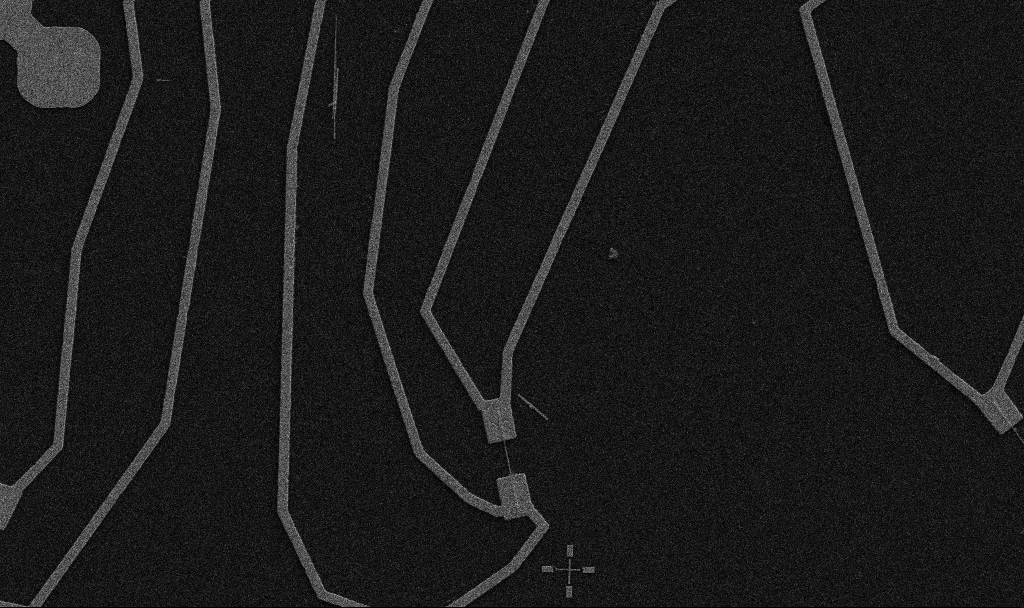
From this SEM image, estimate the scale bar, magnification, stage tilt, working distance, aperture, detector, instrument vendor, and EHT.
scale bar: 10000 nm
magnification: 5 K X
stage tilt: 0°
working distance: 10.7 mm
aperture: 30 µm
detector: SE2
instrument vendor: Zeiss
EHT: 5 kV